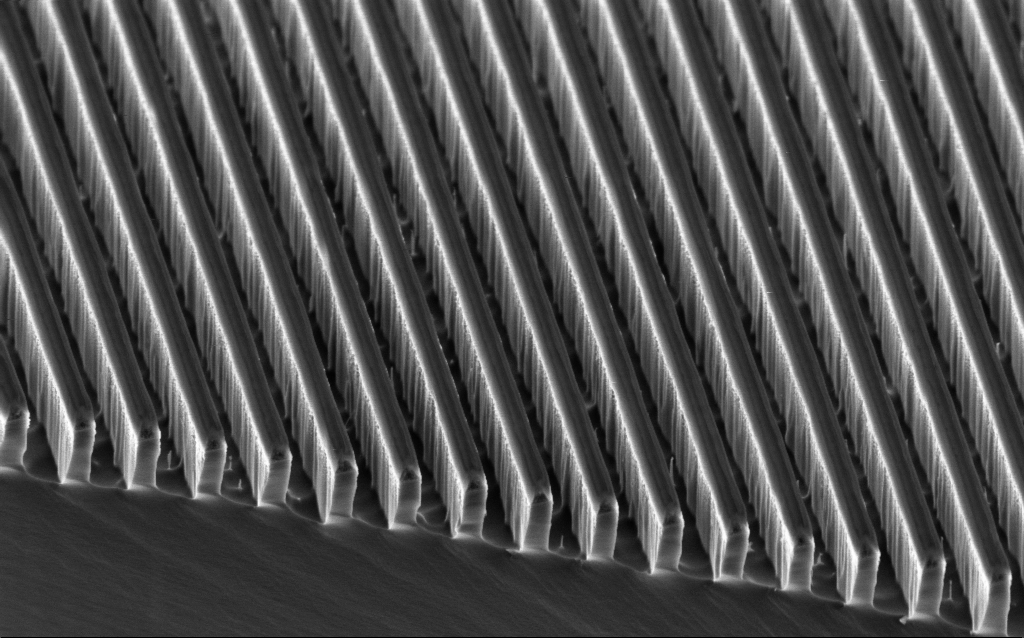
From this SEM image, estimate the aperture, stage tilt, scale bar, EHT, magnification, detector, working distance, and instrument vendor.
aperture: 30 µm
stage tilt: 45°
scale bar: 1000 nm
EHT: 2 kV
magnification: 43 K X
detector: InLens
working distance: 3.6 mm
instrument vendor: Zeiss